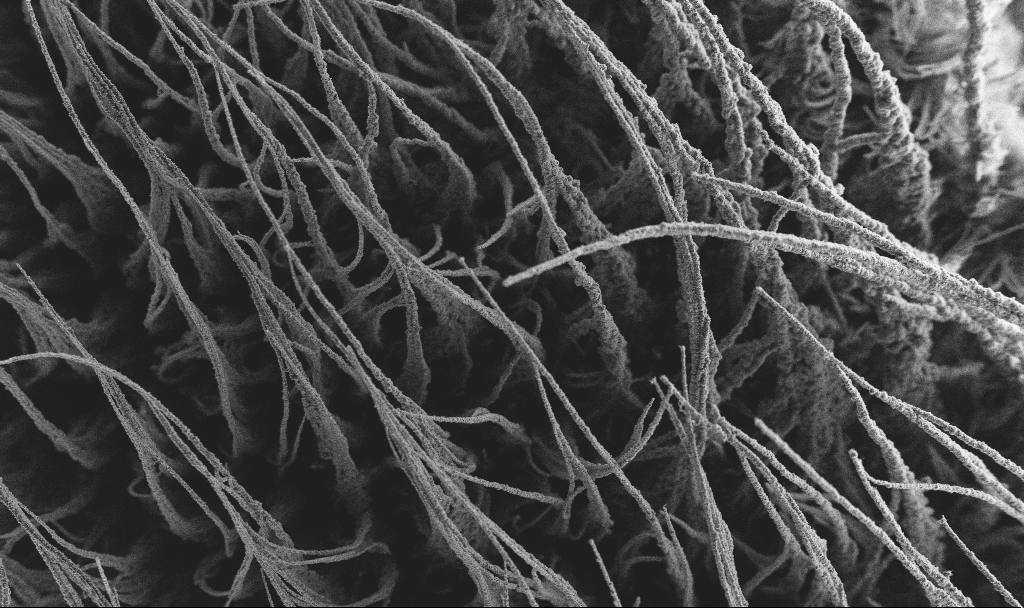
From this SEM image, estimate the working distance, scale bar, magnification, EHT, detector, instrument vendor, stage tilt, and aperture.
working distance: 2.9 mm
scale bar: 100000 nm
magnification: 0.15 K X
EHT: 3 kV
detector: SE2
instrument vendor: Zeiss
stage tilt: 0°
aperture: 30 µm